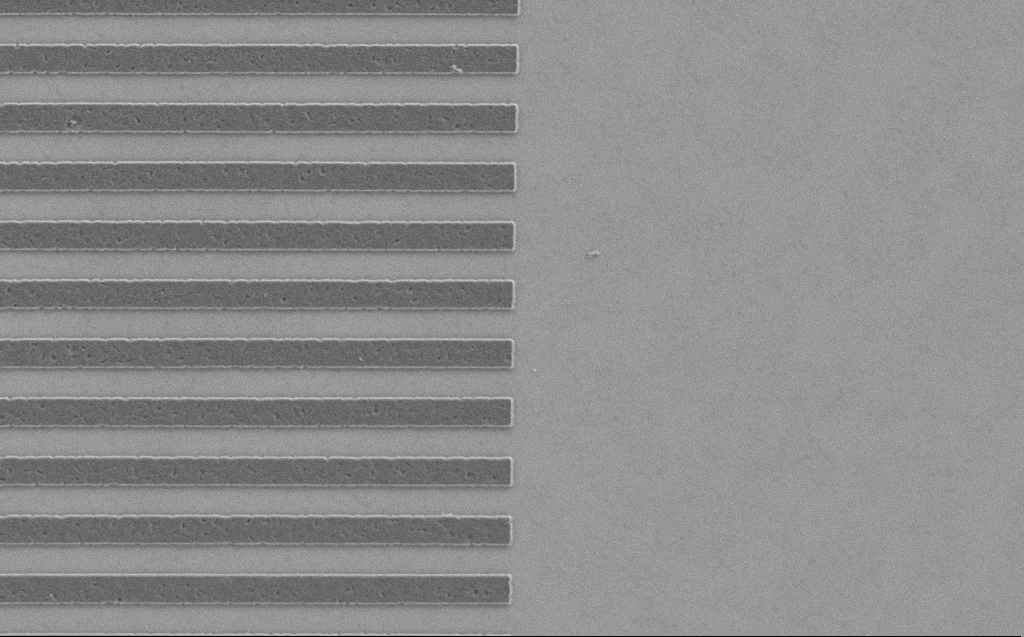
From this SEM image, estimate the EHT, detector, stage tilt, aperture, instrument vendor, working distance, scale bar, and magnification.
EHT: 1.2 kV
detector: SE2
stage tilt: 0°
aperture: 30 µm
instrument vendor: Zeiss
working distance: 5 mm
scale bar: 2000 nm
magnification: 13.63 K X